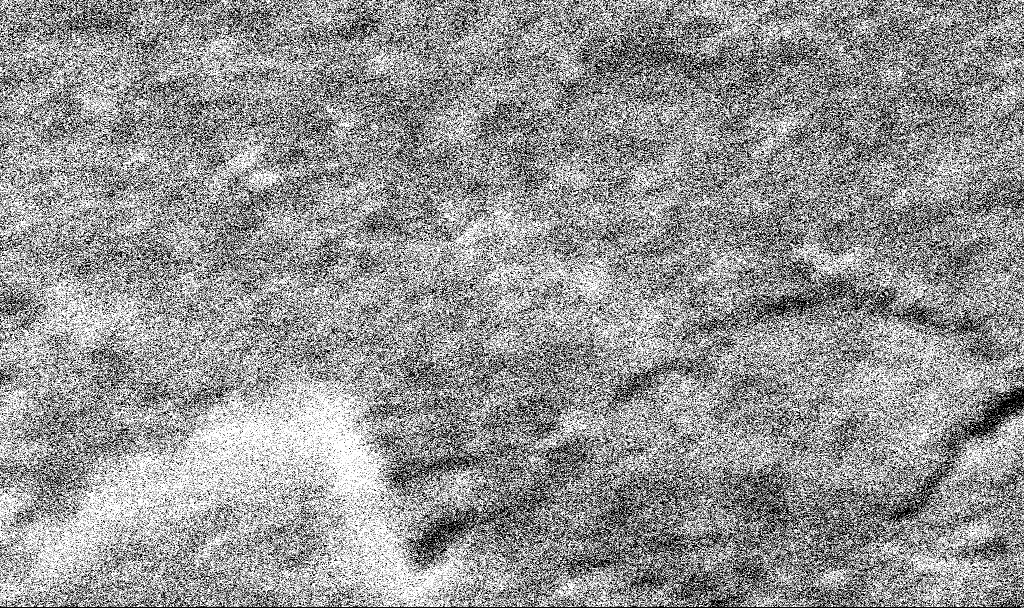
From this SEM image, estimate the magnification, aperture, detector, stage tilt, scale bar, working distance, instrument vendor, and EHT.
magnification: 8.81 K X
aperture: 30 µm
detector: SE2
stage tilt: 0°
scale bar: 2000 nm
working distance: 8.5 mm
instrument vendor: Zeiss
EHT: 20 kV